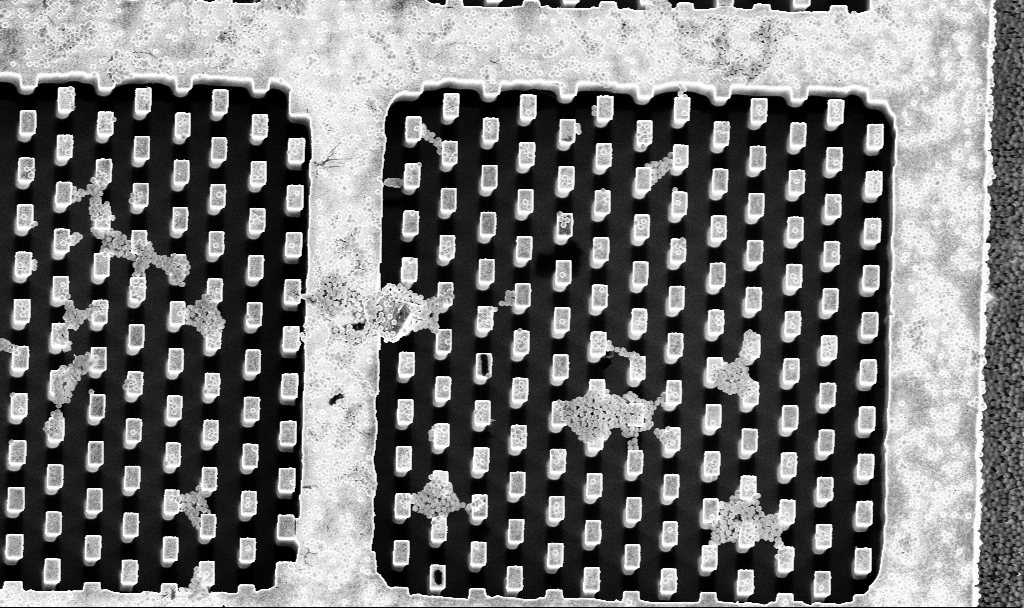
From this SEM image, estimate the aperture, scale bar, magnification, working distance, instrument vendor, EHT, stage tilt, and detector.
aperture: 30 µm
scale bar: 10000 nm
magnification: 3.59 K X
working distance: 3.3 mm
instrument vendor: Zeiss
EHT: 5 kV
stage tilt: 5°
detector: InLens